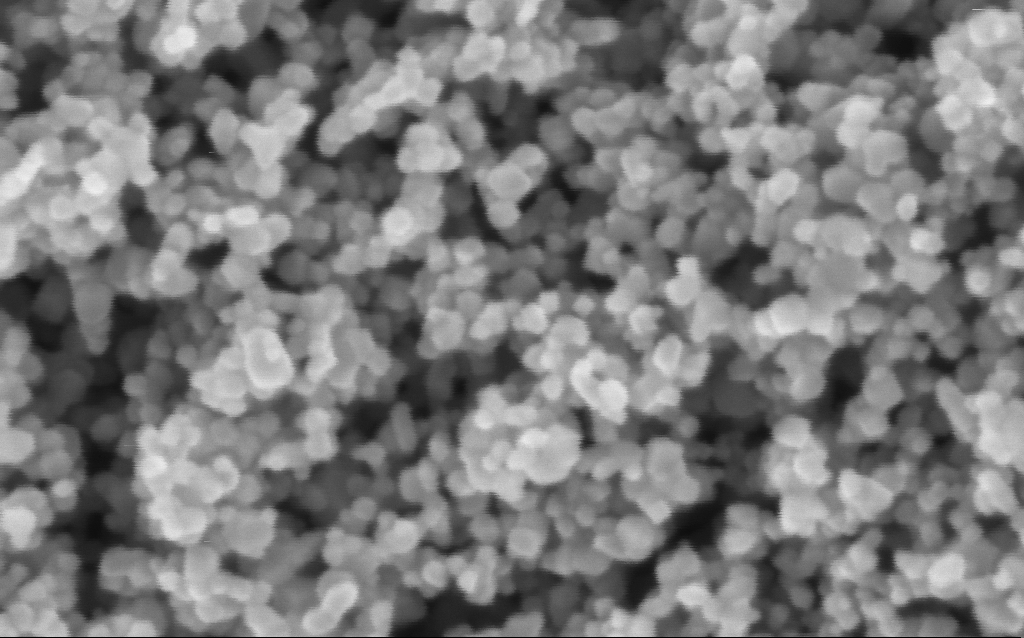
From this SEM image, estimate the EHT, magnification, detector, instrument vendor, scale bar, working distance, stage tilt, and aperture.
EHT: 3 kV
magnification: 416 K X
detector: InLens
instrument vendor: Zeiss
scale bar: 100 nm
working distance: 7.6 mm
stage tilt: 0°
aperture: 30 µm